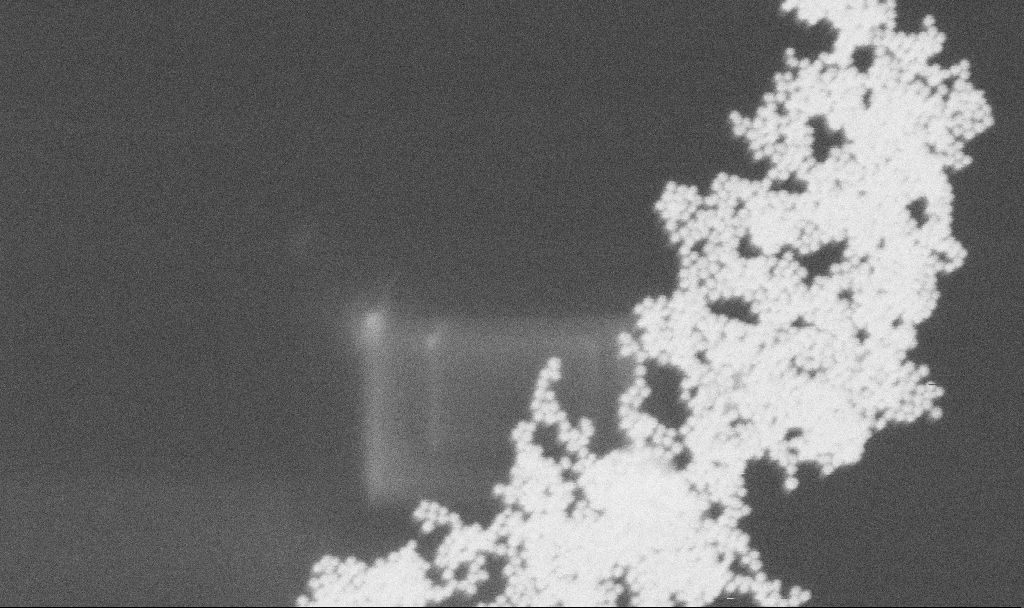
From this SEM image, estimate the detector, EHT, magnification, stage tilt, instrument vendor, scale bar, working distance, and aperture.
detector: SE2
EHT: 15 kV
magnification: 200 K X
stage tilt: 0°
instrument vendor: Zeiss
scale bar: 100 nm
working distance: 11.3 mm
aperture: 30 µm